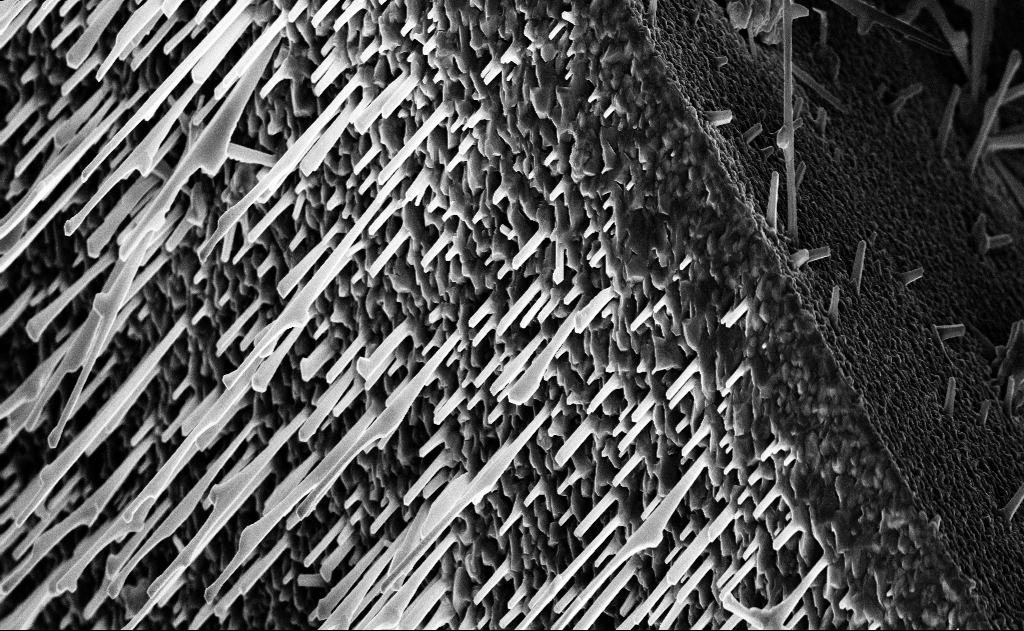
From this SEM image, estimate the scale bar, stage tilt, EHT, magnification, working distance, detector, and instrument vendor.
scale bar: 2000 nm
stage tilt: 0°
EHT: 10 kV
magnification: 10 K X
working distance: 15 mm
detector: InLens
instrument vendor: Zeiss